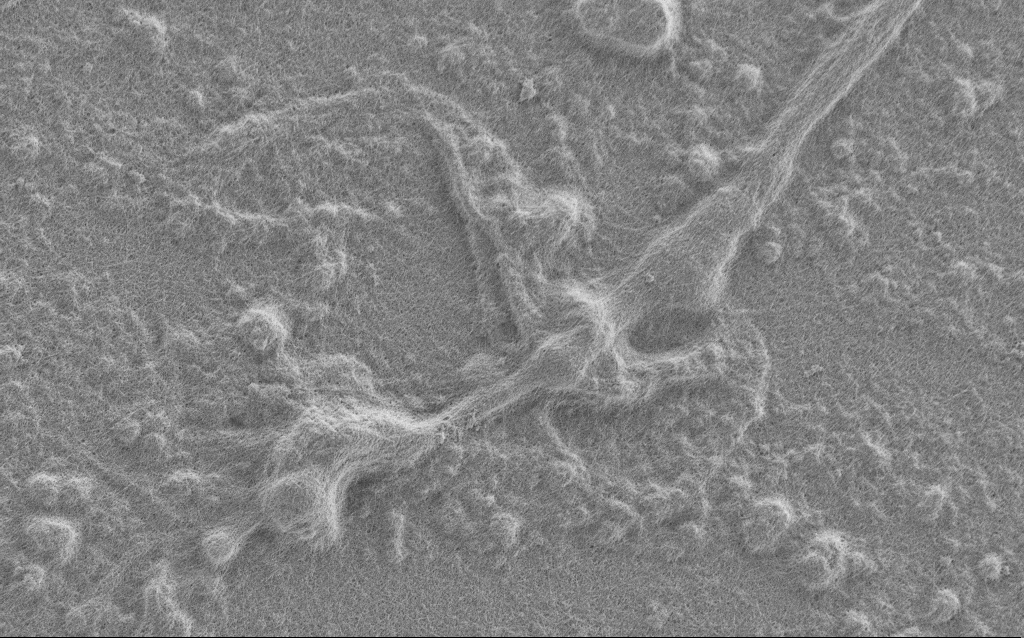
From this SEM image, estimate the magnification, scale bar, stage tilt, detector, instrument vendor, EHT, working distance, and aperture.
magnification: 7.5 K X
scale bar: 2000 nm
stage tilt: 0°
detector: SE2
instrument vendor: Zeiss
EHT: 1 kV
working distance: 6 mm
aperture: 30 µm